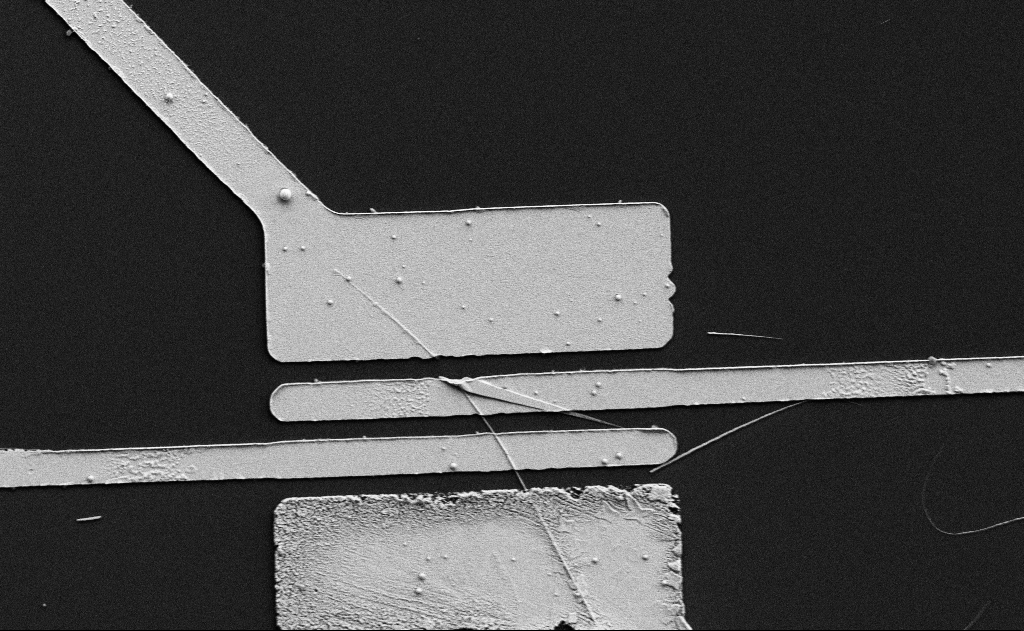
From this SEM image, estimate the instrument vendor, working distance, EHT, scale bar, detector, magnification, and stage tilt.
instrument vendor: Zeiss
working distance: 15 mm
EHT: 5 kV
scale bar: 10000 nm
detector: SE2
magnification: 5 K X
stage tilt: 0°